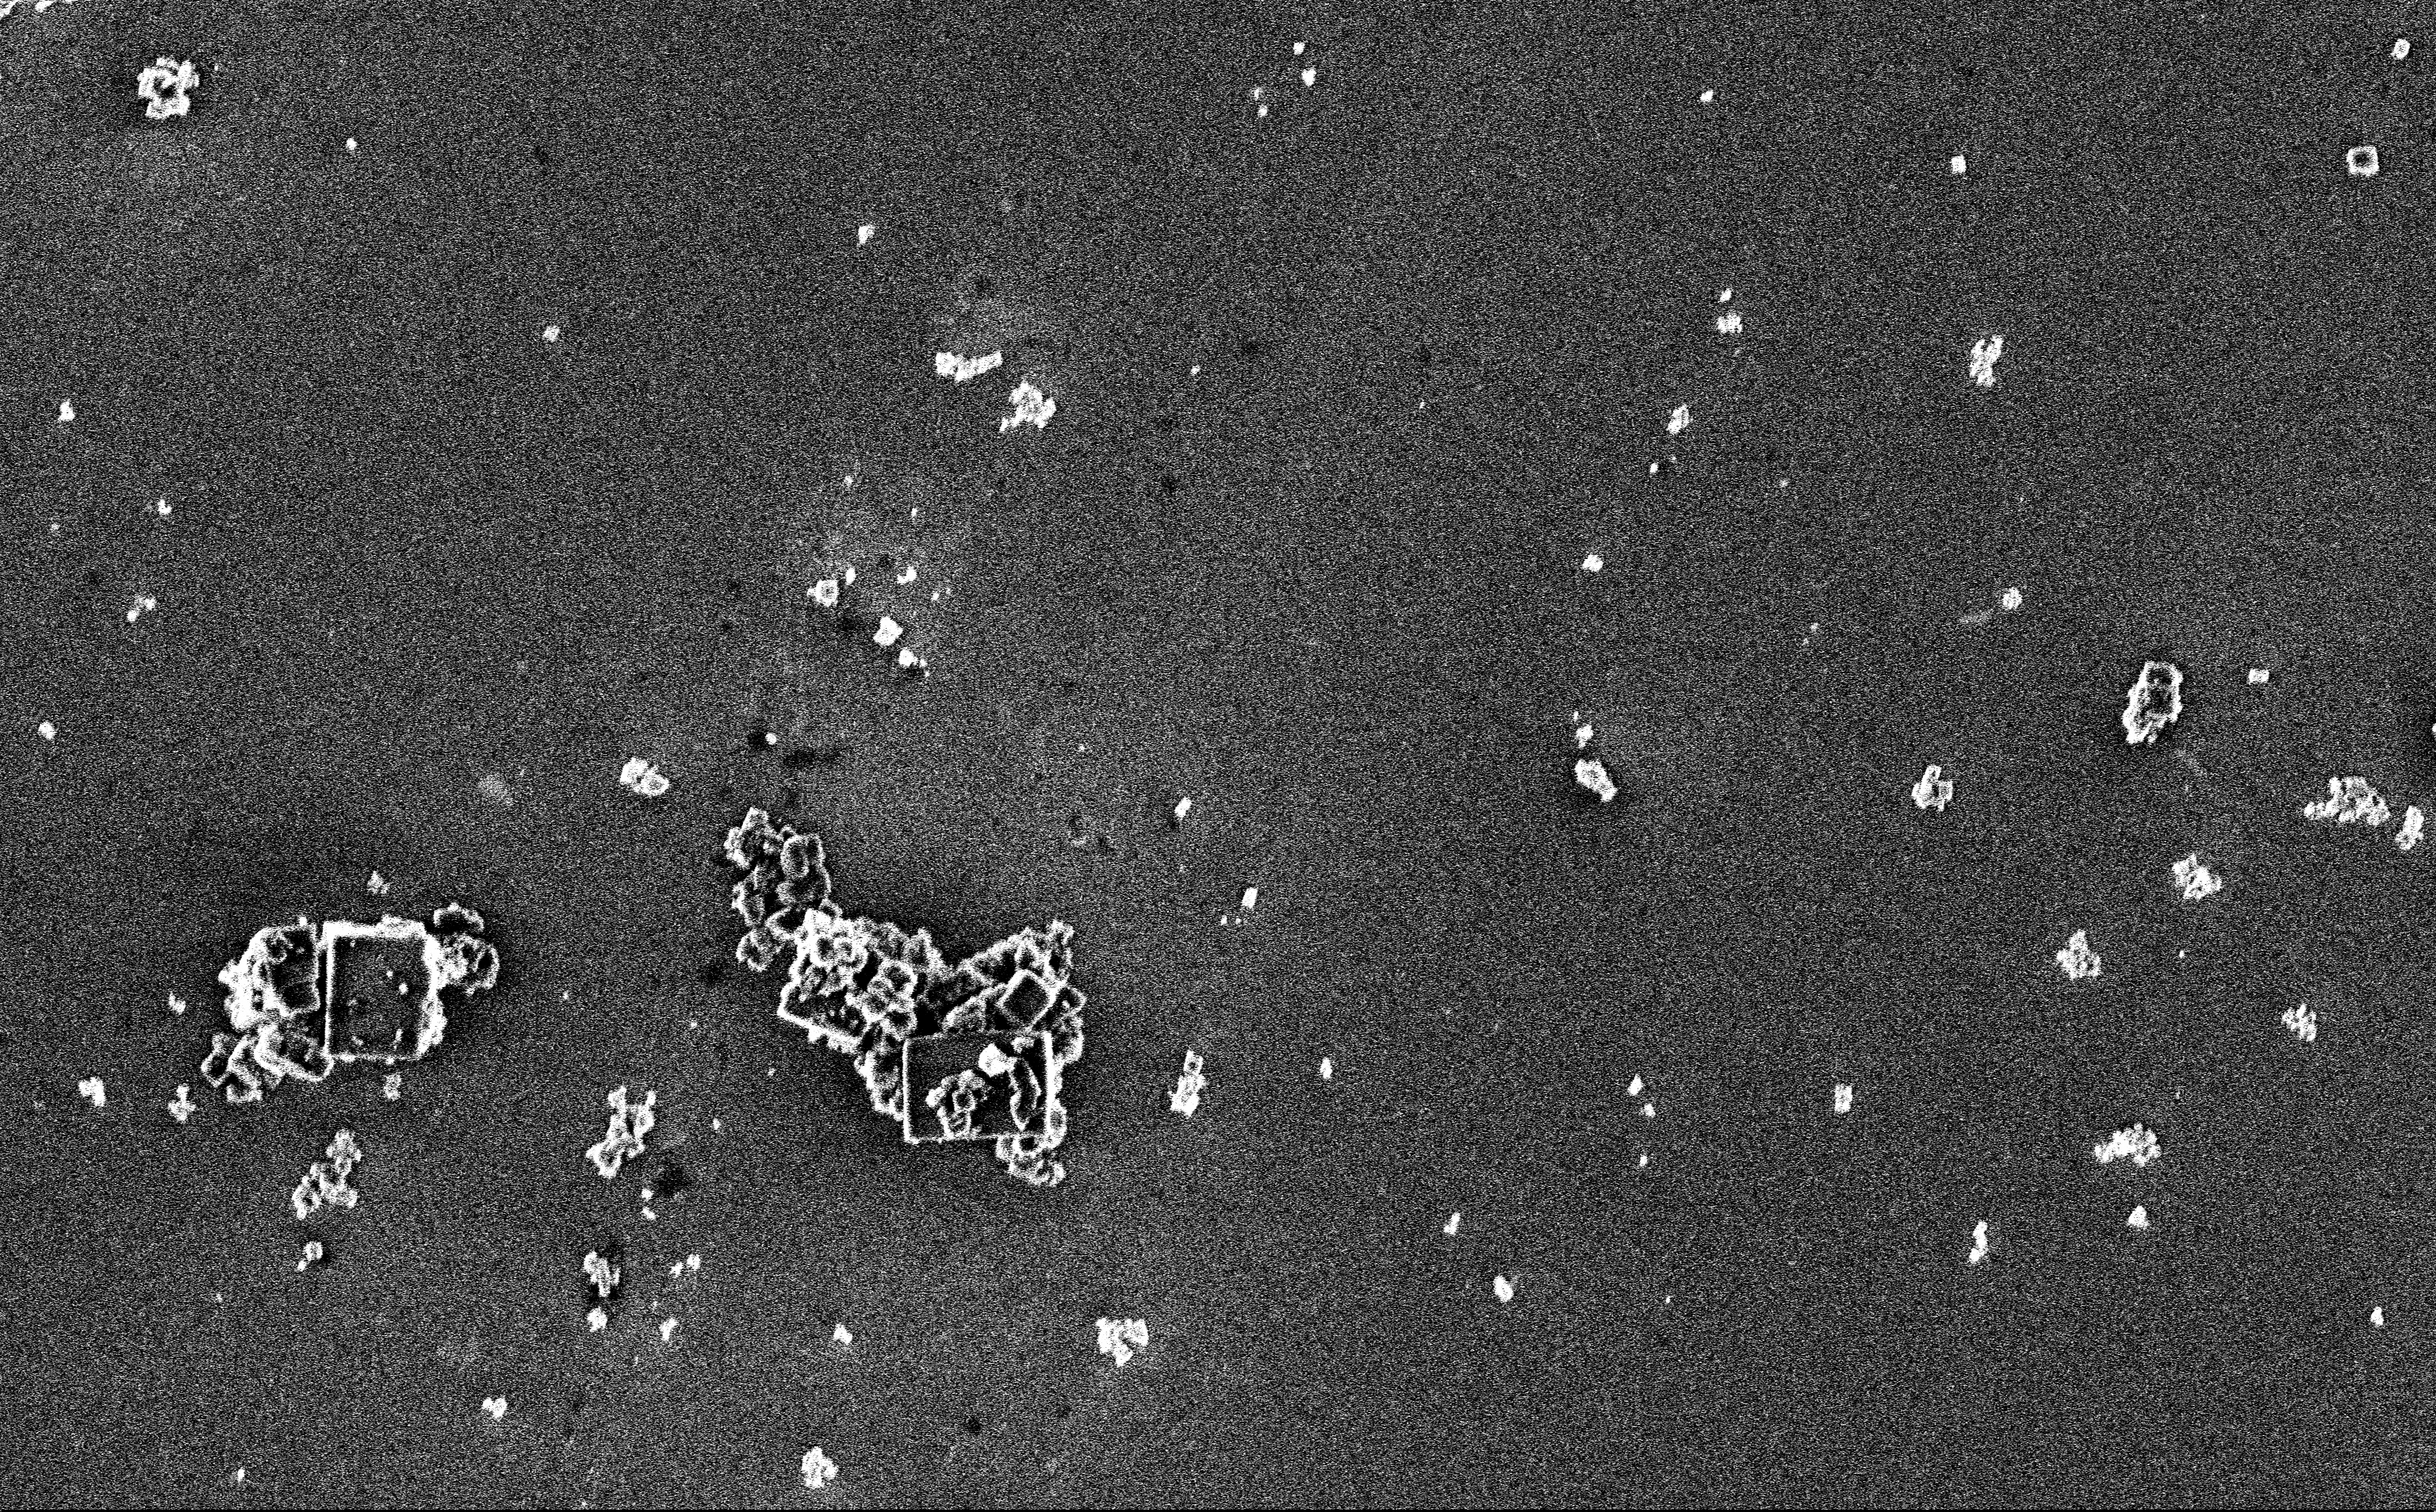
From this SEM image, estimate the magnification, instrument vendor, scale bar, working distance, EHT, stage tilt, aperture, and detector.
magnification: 9.98 K X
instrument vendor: Zeiss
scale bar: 2000 nm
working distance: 3 mm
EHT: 3 kV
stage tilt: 0°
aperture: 30 µm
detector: InLens